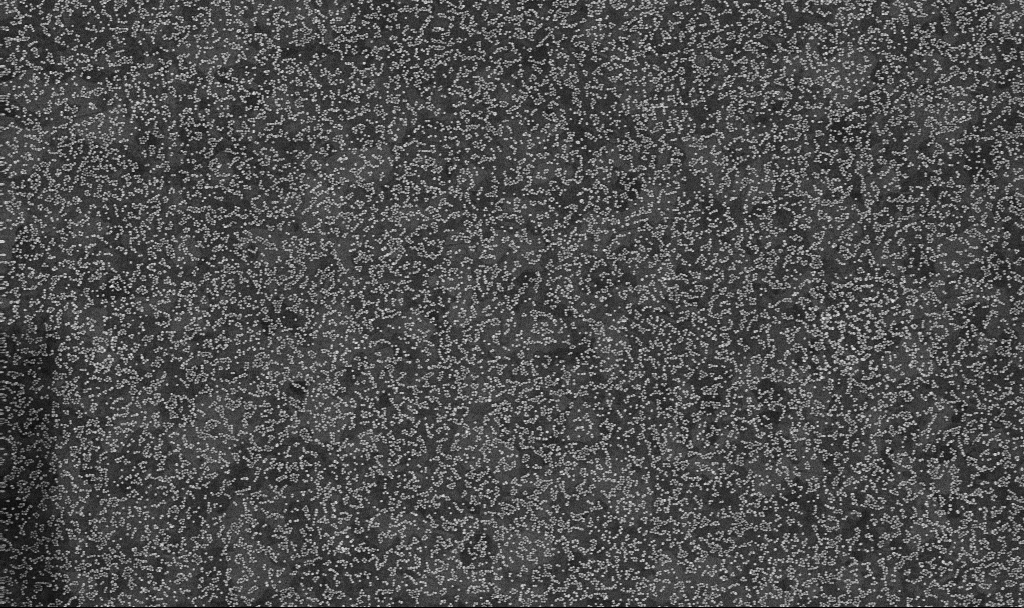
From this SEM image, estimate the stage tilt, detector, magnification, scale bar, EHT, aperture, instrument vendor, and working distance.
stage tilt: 0°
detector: InLens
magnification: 50 K X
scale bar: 1000 nm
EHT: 10 kV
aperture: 30 µm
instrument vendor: Zeiss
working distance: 3.3 mm